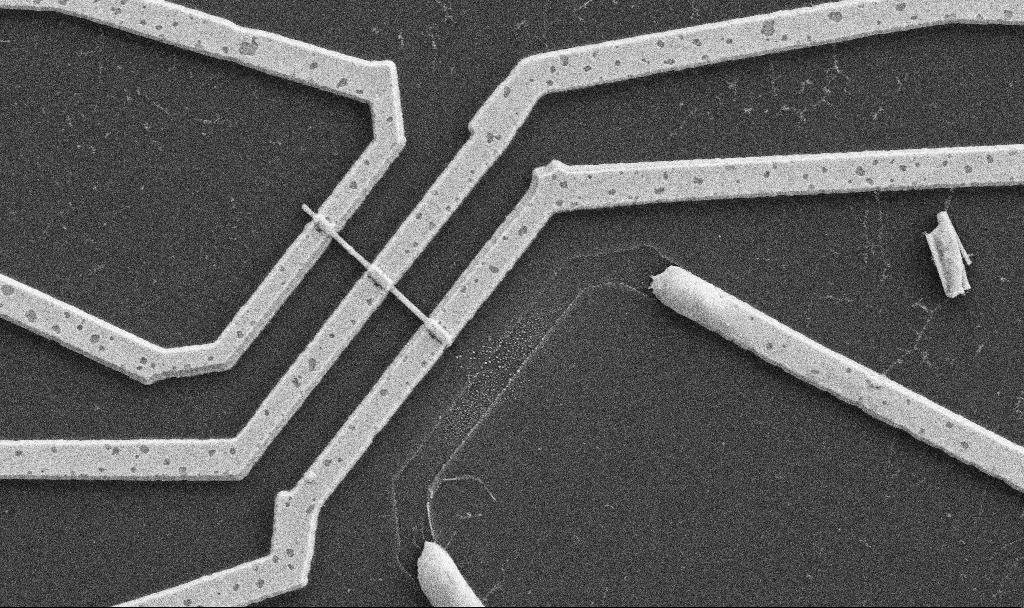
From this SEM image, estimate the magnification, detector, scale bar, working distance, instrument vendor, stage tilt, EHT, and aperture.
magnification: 20 K X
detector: SE2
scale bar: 1000 nm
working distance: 10.7 mm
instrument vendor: Zeiss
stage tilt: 0°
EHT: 5 kV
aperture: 30 µm